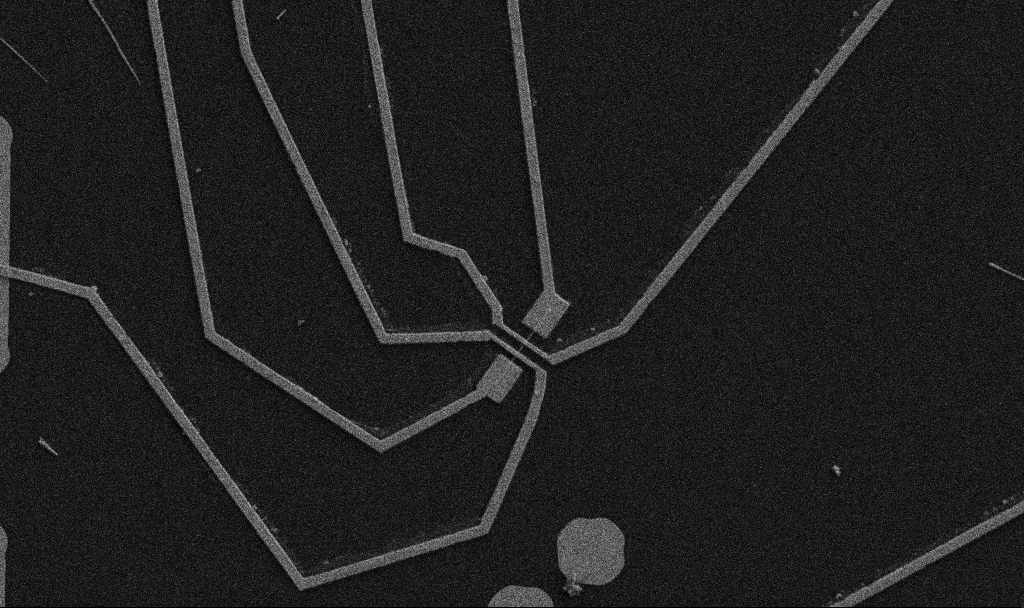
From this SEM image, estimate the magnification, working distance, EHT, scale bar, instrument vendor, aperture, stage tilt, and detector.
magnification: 5 K X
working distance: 10.7 mm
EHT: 5 kV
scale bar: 10000 nm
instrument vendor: Zeiss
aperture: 30 µm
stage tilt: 0°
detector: SE2